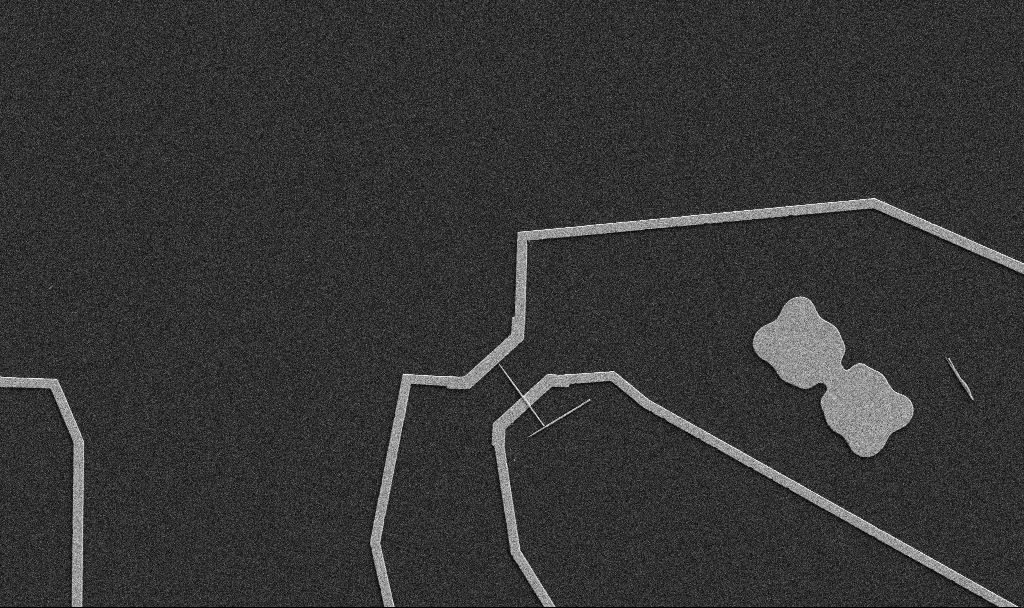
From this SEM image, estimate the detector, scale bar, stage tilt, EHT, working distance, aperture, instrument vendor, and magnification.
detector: SE2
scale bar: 10000 nm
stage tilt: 0°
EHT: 5 kV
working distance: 10.7 mm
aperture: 30 µm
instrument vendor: Zeiss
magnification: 5 K X